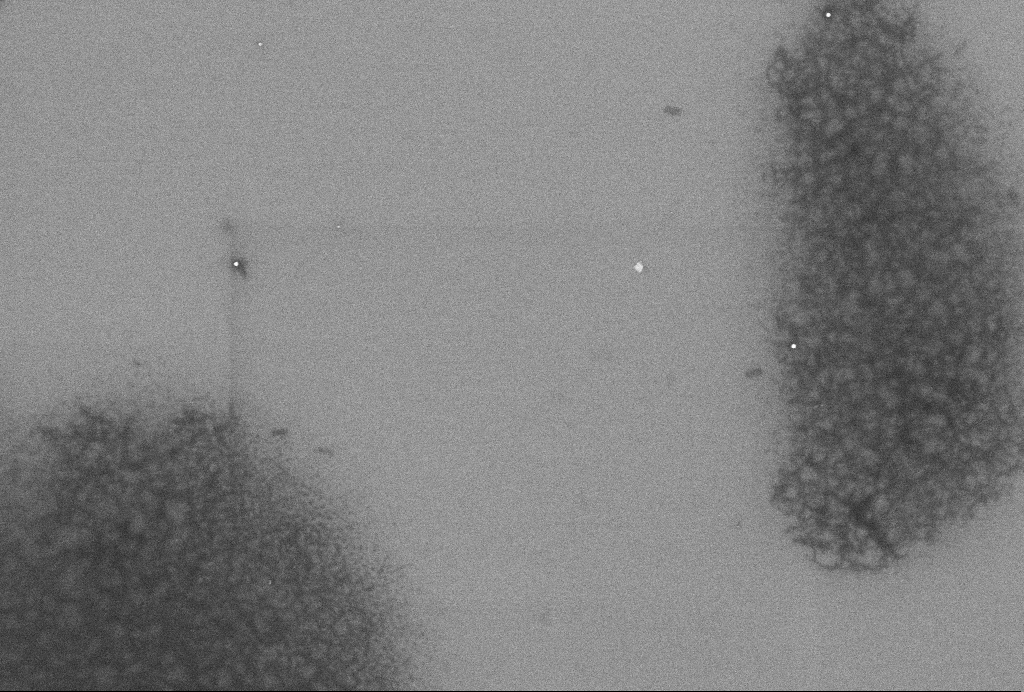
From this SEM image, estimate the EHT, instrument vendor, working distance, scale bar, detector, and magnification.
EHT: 2 kV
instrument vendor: Zeiss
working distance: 3.3 mm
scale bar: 1000 nm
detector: InLens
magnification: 54.42 K X